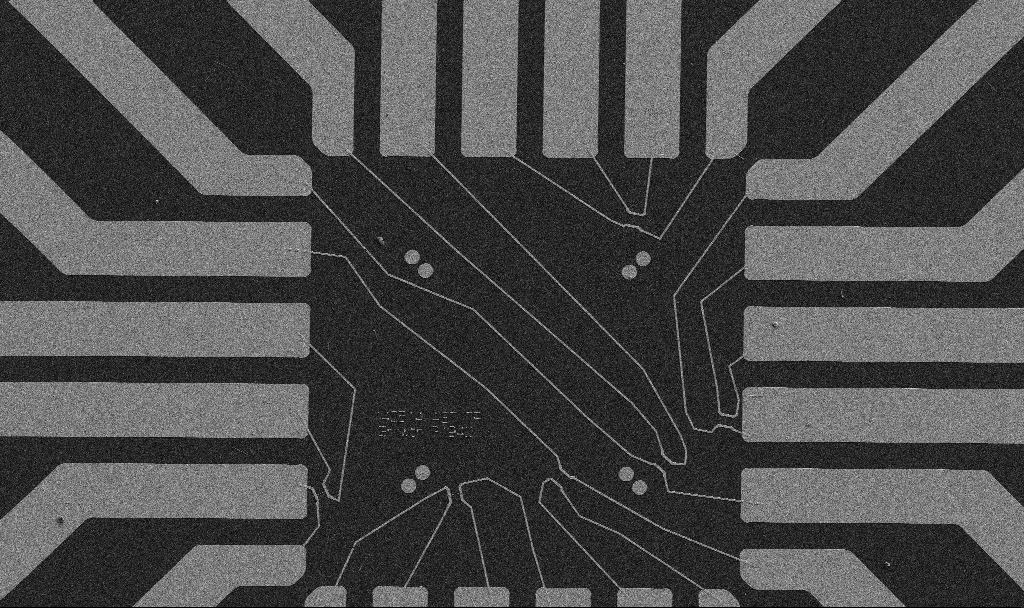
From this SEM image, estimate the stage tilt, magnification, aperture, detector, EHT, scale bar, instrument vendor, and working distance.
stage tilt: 0°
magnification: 1 K X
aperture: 30 µm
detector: SE2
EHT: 5 kV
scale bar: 20000 nm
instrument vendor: Zeiss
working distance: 10.7 mm